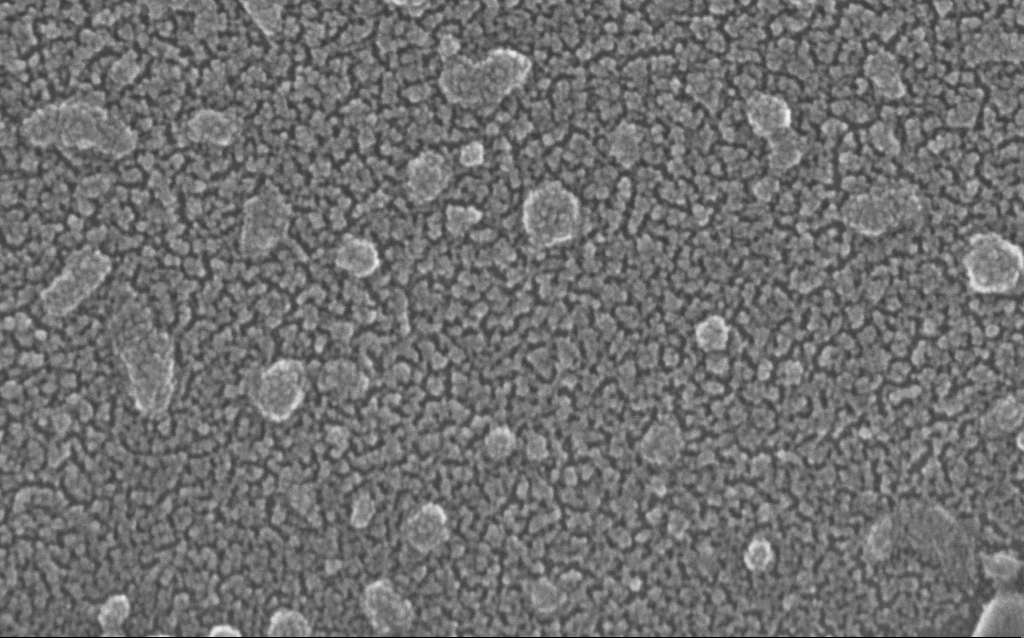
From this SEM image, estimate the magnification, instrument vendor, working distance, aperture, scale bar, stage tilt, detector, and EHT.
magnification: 500 K X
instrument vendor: Zeiss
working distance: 1.5 mm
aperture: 30 µm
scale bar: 100 nm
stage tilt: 0°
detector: InLens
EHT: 20 kV